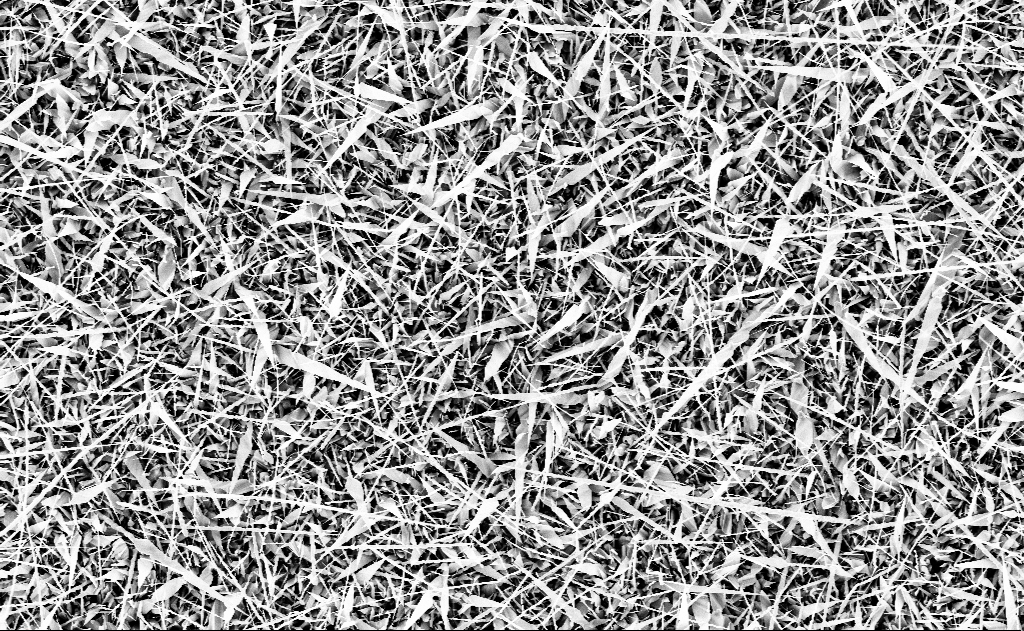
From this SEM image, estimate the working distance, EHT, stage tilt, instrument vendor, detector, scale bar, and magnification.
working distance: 13 mm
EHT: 10 kV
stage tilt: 0°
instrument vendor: Zeiss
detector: InLens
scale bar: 10000 nm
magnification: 5 K X